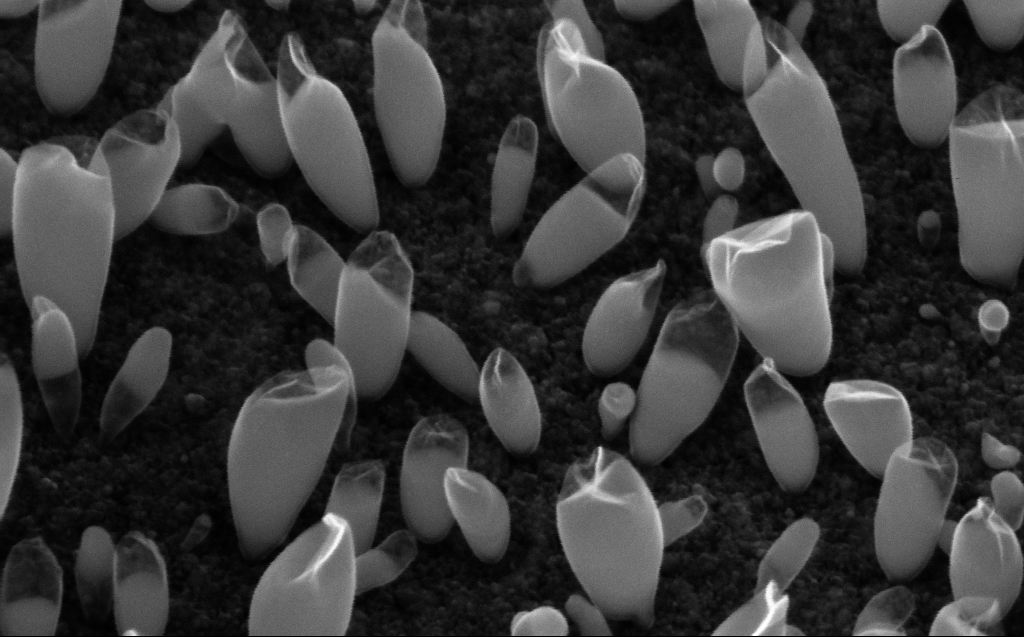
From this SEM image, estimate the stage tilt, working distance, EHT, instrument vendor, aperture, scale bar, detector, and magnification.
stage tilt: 45°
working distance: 5 mm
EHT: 10 kV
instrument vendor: Zeiss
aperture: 30 µm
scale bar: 100 nm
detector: InLens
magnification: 200 K X